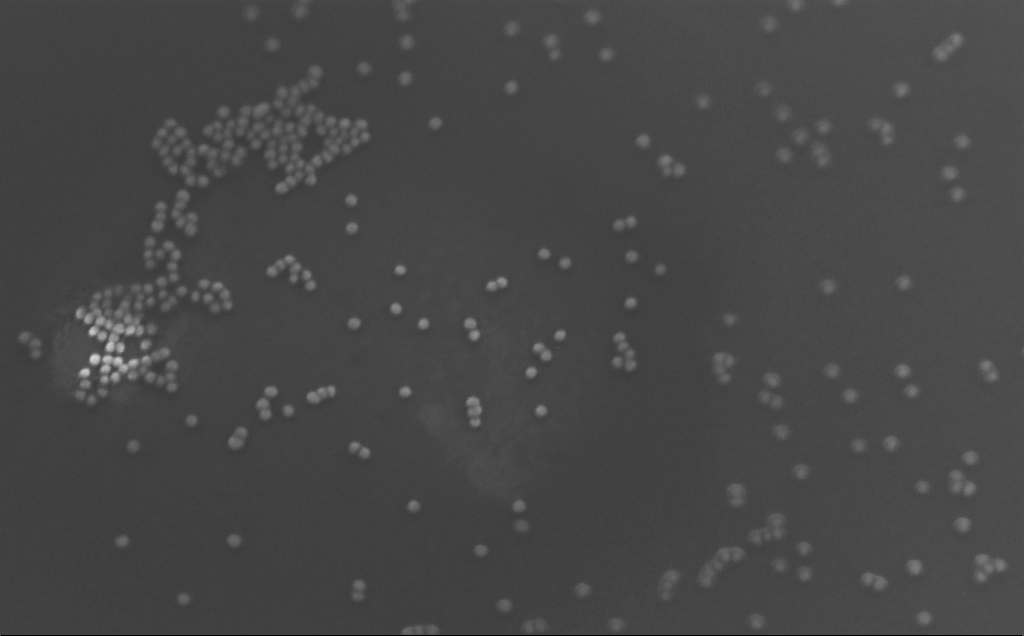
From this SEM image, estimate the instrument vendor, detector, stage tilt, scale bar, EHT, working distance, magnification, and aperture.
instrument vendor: Zeiss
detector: InLens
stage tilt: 25°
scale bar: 100 nm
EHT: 10 kV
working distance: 4 mm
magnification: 241.11 K X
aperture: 30 µm